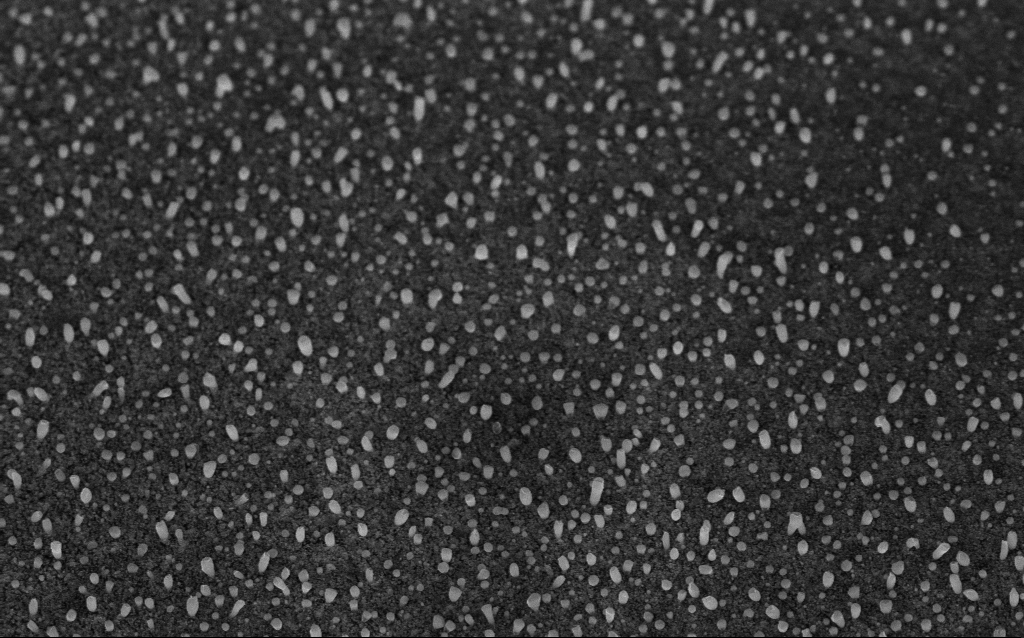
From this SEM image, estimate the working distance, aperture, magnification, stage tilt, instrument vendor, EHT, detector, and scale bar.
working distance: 5.4 mm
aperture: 30 µm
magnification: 50 K X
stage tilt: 45°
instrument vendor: Zeiss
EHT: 5 kV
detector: InLens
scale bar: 1000 nm